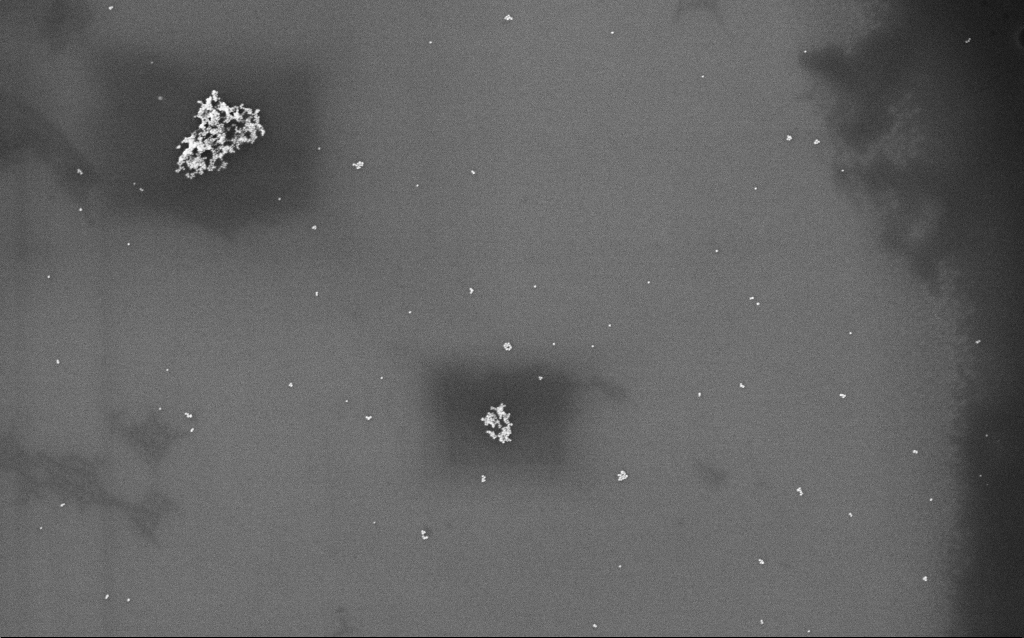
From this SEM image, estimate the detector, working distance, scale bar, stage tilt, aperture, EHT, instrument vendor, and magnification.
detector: InLens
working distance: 2.9 mm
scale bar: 1000 nm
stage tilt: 0°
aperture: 30 µm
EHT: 4 kV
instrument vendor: Zeiss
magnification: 48.81 K X